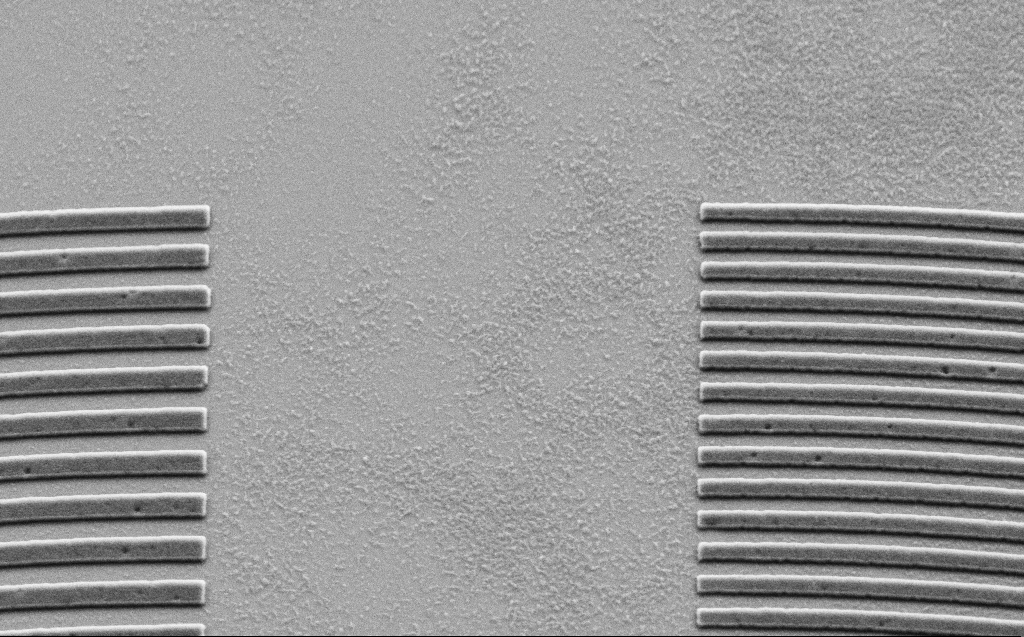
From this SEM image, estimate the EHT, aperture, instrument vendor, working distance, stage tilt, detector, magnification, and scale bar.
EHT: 2.5 kV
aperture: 30 µm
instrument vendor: Zeiss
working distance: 5 mm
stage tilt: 30°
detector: SE2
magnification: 18.67 K X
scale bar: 2000 nm